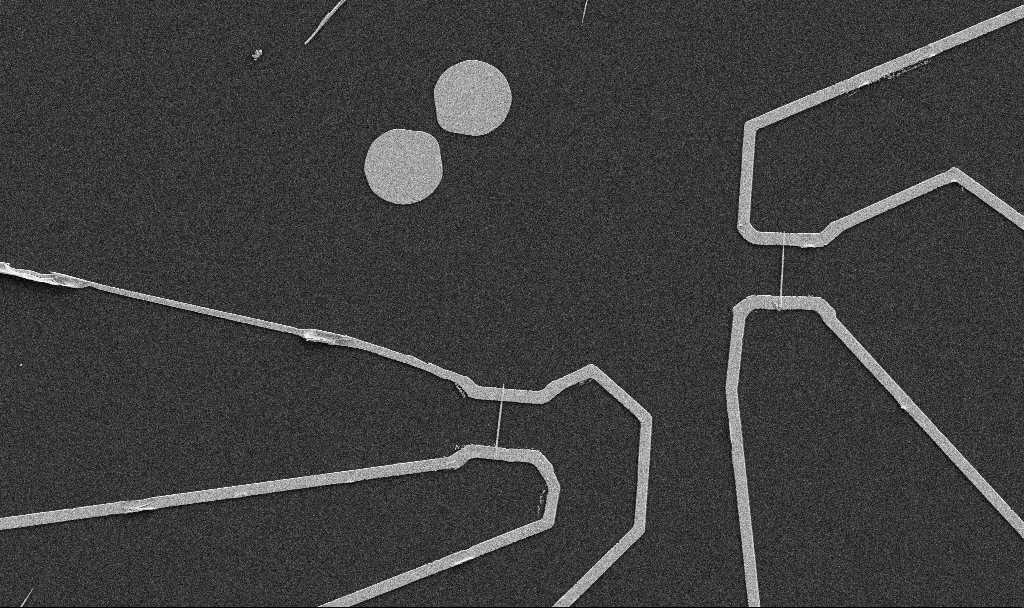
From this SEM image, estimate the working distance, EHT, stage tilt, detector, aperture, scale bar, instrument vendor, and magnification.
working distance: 10.7 mm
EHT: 5 kV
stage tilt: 0°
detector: SE2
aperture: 30 µm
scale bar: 10000 nm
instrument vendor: Zeiss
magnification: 5 K X